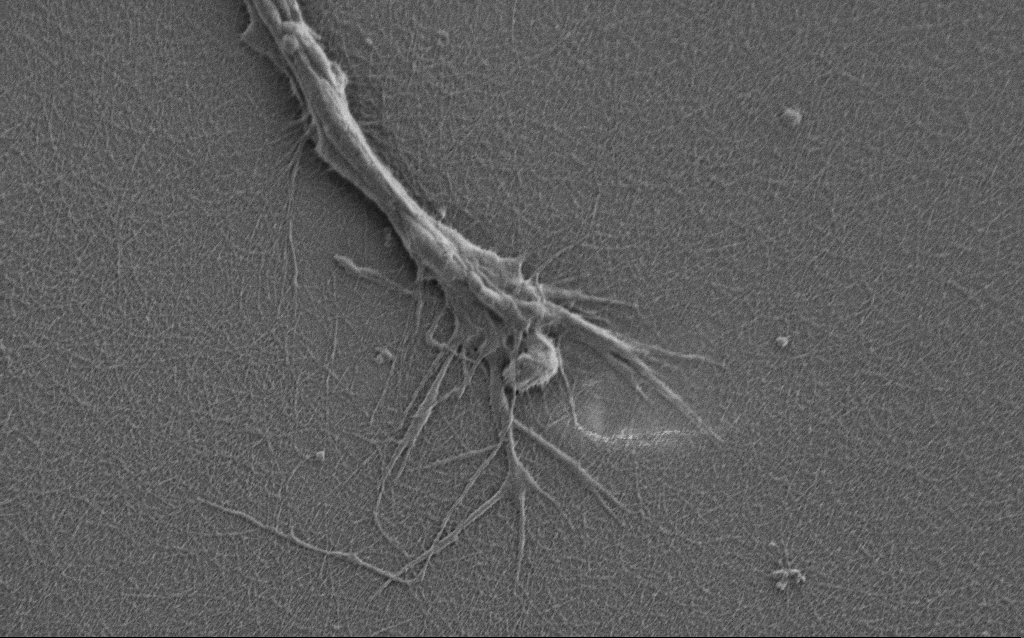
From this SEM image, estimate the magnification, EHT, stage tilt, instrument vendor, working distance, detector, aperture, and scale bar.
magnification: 10 K X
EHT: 0.9 kV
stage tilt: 0°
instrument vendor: Zeiss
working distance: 7 mm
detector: SE2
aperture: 30 µm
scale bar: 2000 nm